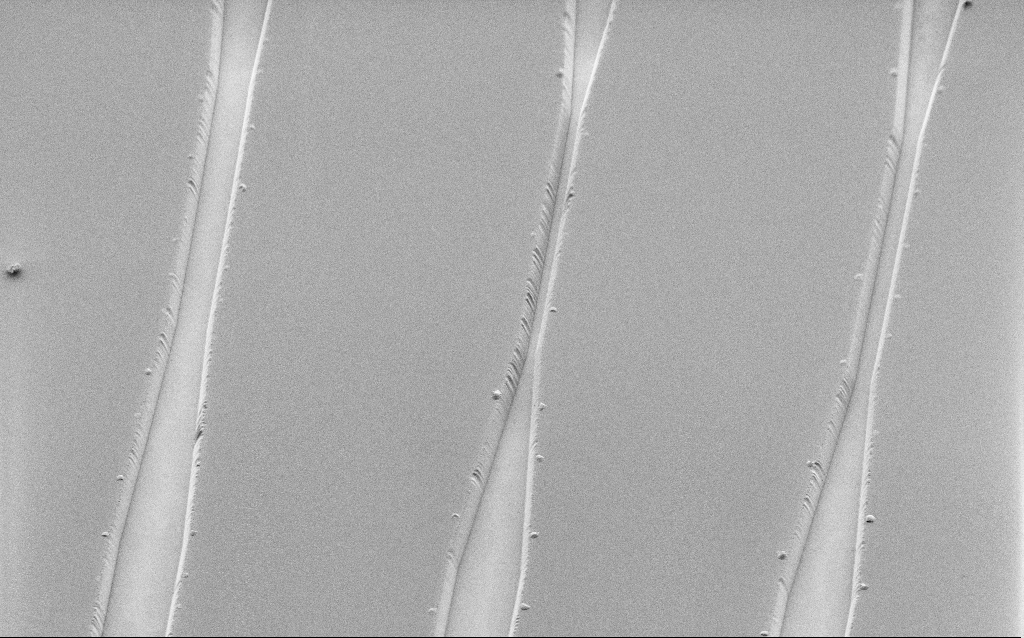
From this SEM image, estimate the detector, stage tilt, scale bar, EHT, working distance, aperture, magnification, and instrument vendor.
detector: SE2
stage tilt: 45°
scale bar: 20000 nm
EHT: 1.2 kV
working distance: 8 mm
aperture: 30 µm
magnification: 1.18 K X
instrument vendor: Zeiss